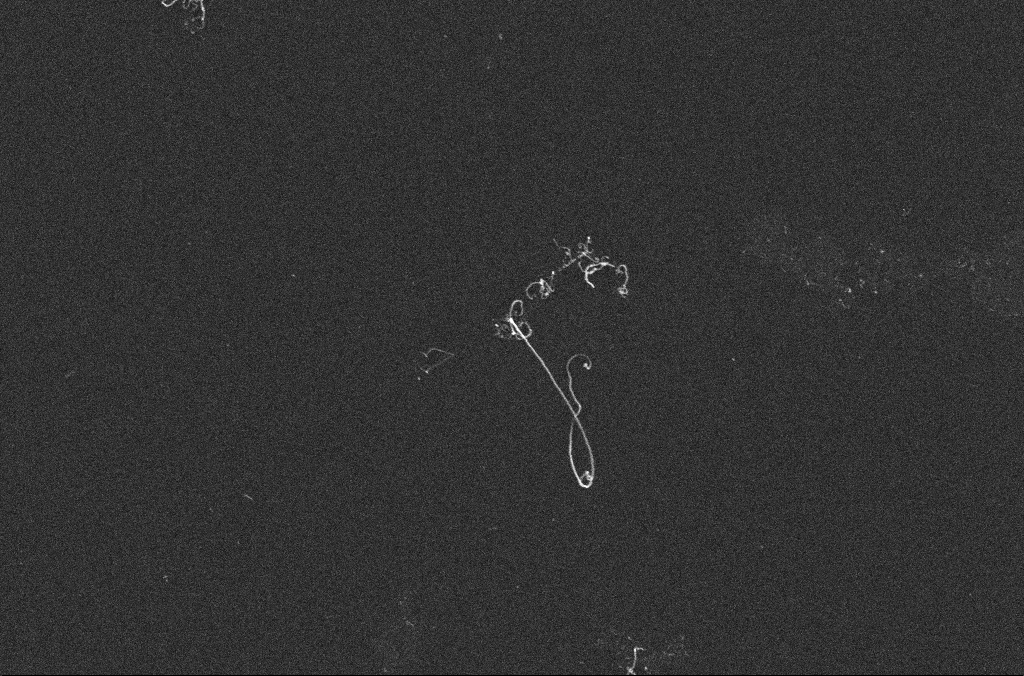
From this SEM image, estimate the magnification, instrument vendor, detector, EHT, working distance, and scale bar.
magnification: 50 K X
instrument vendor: Zeiss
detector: InLens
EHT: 10 kV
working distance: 3.3 mm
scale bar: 1000 nm